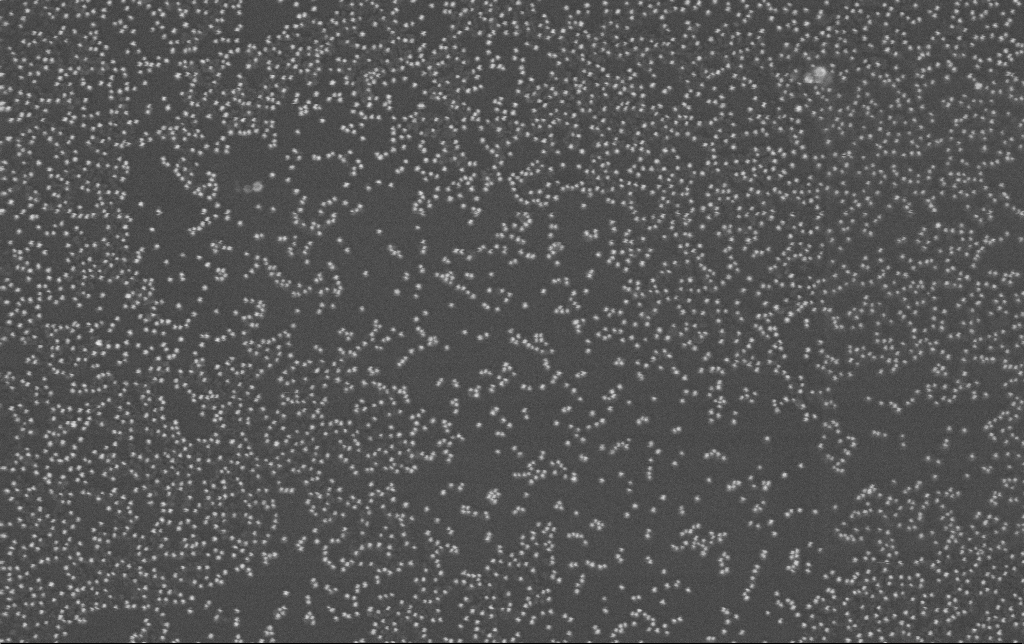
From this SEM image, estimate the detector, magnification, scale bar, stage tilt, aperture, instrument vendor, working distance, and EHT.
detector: InLens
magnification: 109.29 K X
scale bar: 200 nm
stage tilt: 0°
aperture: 30 µm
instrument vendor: Zeiss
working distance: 11.3 mm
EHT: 10 kV